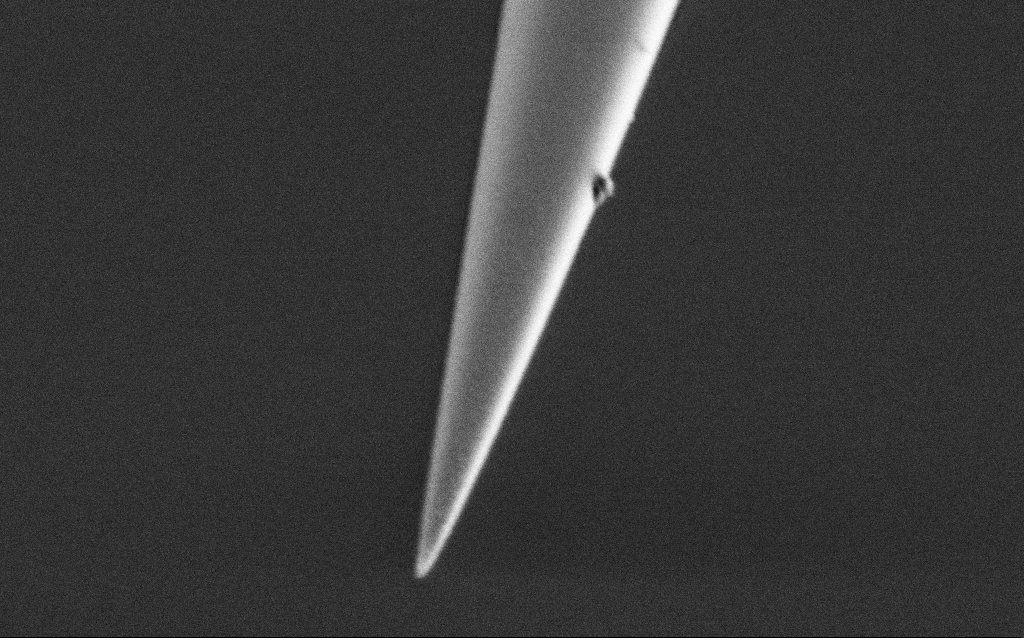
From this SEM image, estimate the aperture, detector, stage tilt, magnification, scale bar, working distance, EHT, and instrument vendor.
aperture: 30 µm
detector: SE2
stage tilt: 45°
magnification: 50 K X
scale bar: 1000 nm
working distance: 6.6 mm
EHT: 1 kV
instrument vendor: Zeiss